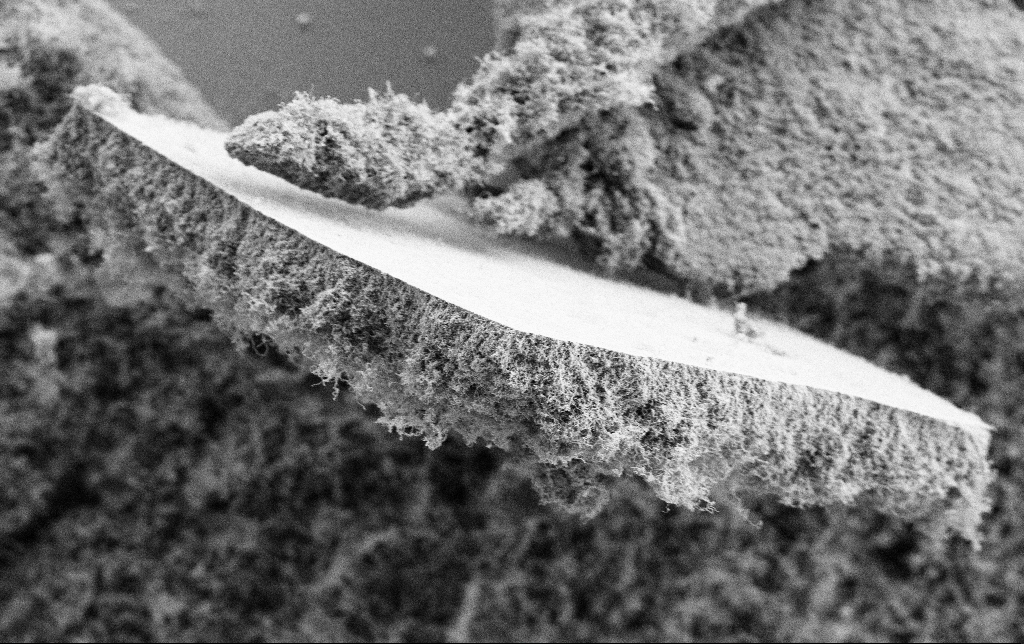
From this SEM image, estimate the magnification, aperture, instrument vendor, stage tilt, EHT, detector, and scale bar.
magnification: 5 K X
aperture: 30 µm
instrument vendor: Zeiss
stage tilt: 0°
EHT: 2 kV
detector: SE2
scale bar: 10000 nm